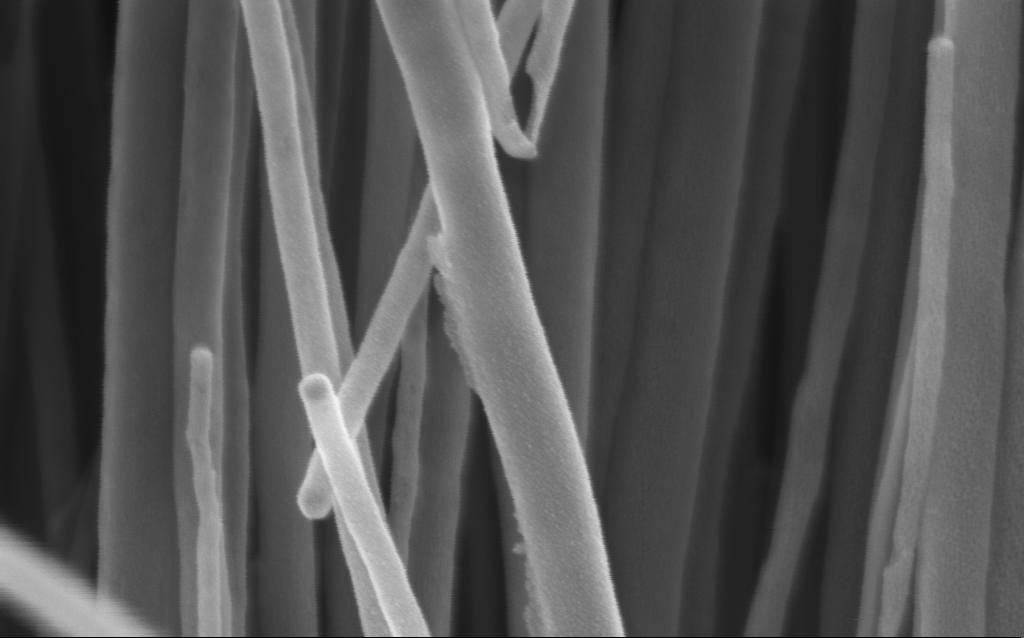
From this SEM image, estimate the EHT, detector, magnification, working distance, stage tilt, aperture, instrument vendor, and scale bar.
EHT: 3 kV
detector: InLens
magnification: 178.04 K X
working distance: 3 mm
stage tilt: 0°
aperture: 30 µm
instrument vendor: Zeiss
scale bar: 200 nm